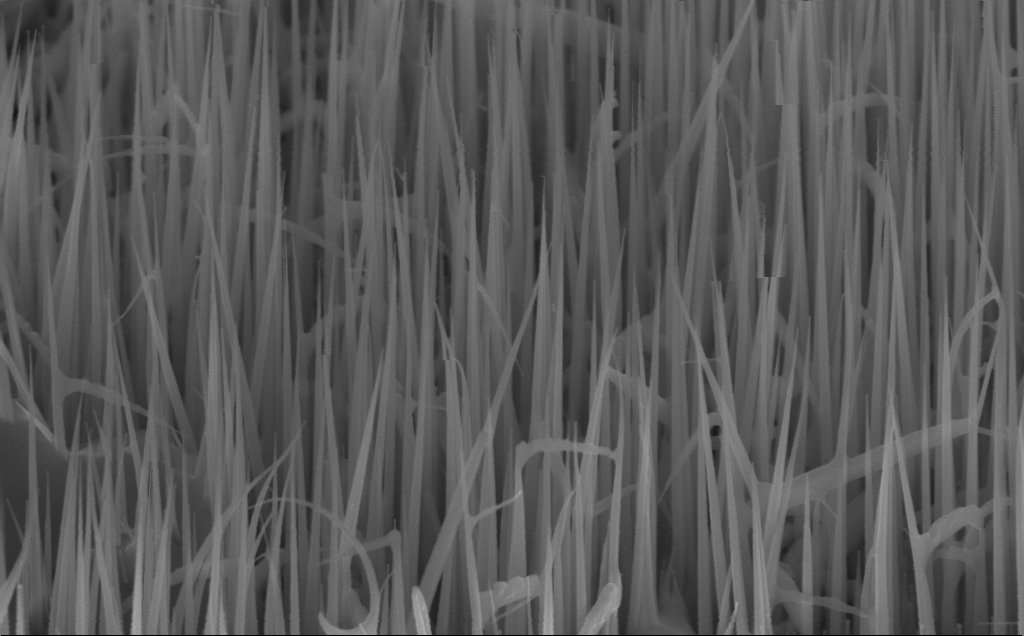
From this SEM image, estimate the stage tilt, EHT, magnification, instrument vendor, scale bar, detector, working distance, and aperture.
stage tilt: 45°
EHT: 10 kV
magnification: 90.89 K X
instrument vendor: Zeiss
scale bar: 200 nm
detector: InLens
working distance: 7 mm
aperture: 30 µm